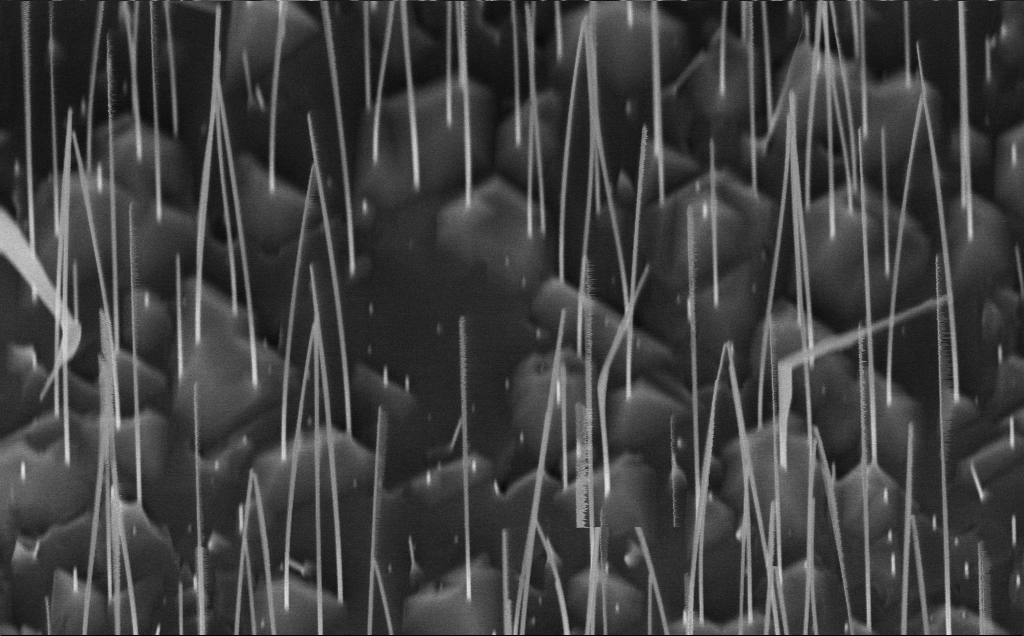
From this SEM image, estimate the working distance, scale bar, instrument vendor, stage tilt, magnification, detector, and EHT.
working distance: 7 mm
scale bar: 1000 nm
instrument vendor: Zeiss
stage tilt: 45°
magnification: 50.78 K X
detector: InLens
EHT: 10 kV